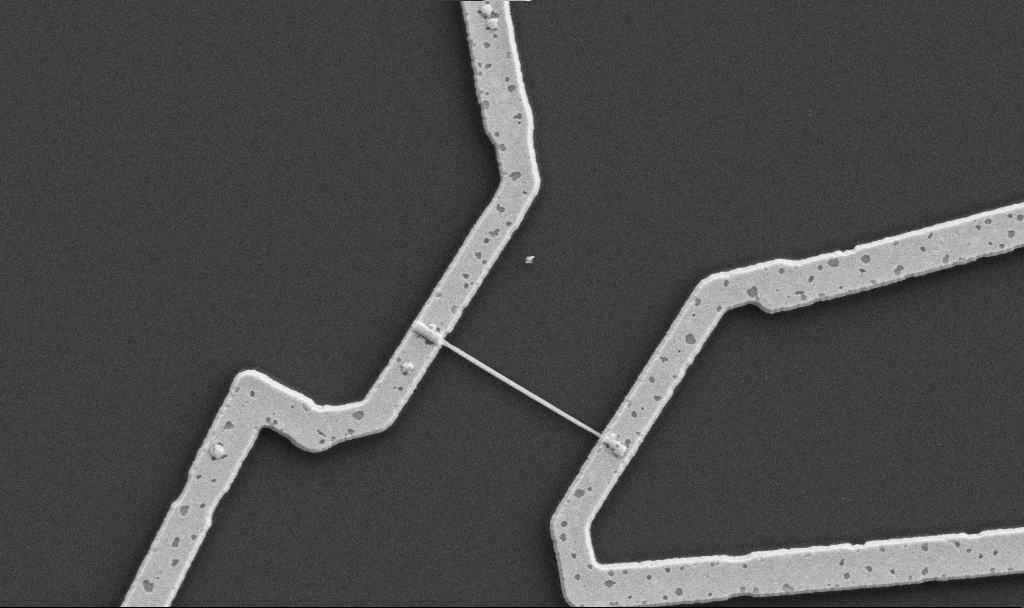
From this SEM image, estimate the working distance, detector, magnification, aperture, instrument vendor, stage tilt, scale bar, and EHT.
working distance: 10.7 mm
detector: SE2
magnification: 20 K X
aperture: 30 µm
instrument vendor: Zeiss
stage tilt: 0°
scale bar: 1000 nm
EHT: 5 kV